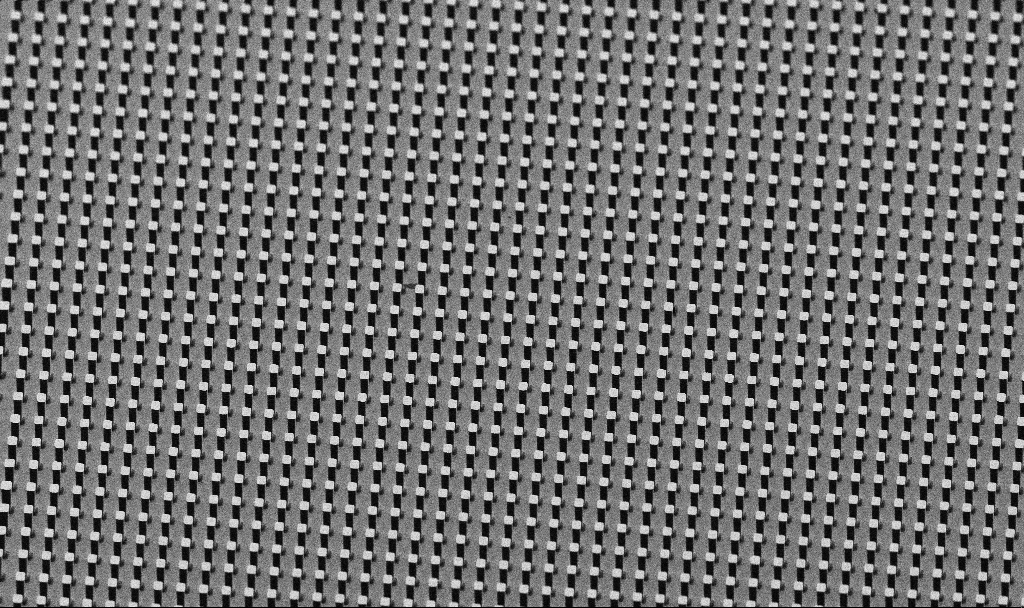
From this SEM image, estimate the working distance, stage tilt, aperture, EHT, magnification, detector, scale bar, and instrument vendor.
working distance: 7.4 mm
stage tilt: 45°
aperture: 30 µm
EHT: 5 kV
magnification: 0.697 K X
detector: SE2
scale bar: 100000 nm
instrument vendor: Zeiss